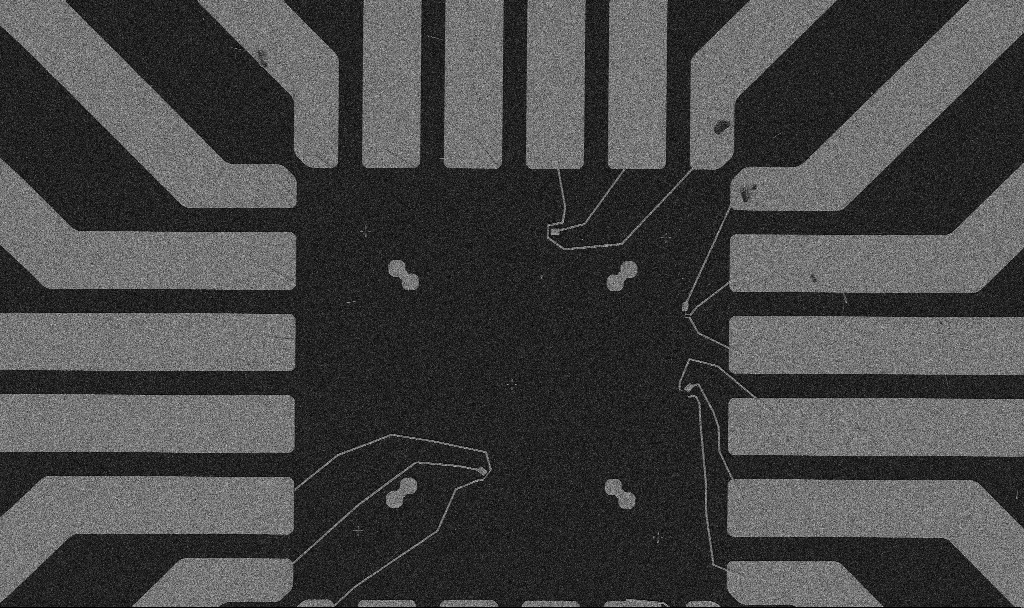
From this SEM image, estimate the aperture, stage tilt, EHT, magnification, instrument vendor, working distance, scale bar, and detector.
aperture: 30 µm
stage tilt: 0°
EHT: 5 kV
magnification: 1 K X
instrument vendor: Zeiss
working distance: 10.7 mm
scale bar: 20000 nm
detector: SE2